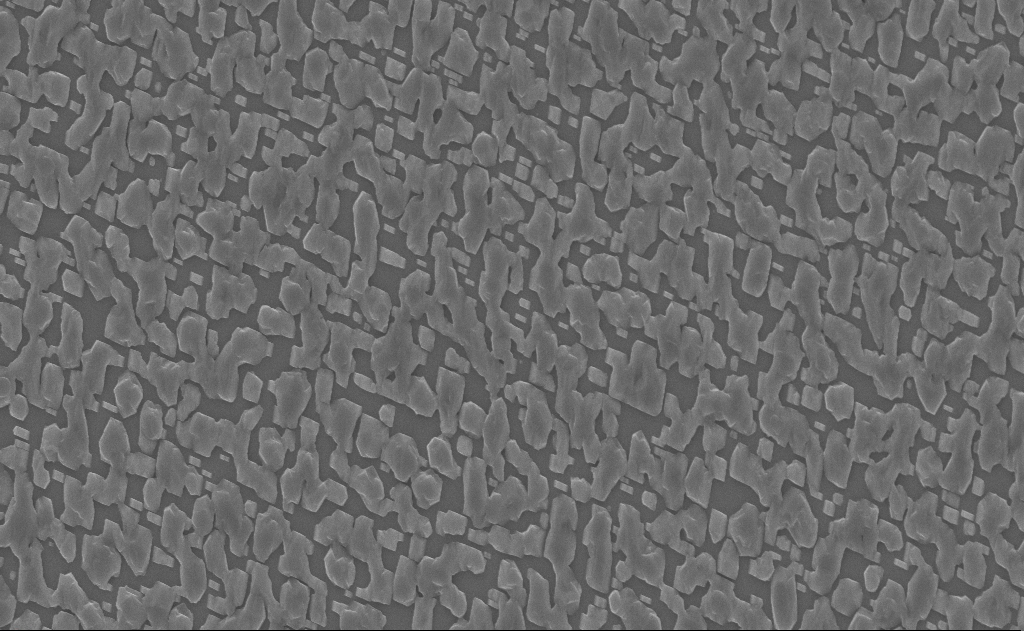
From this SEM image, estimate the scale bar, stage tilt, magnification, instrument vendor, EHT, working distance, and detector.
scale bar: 2000 nm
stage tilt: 0°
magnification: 10 K X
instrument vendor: Zeiss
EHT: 10 kV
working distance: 18 mm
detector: InLens